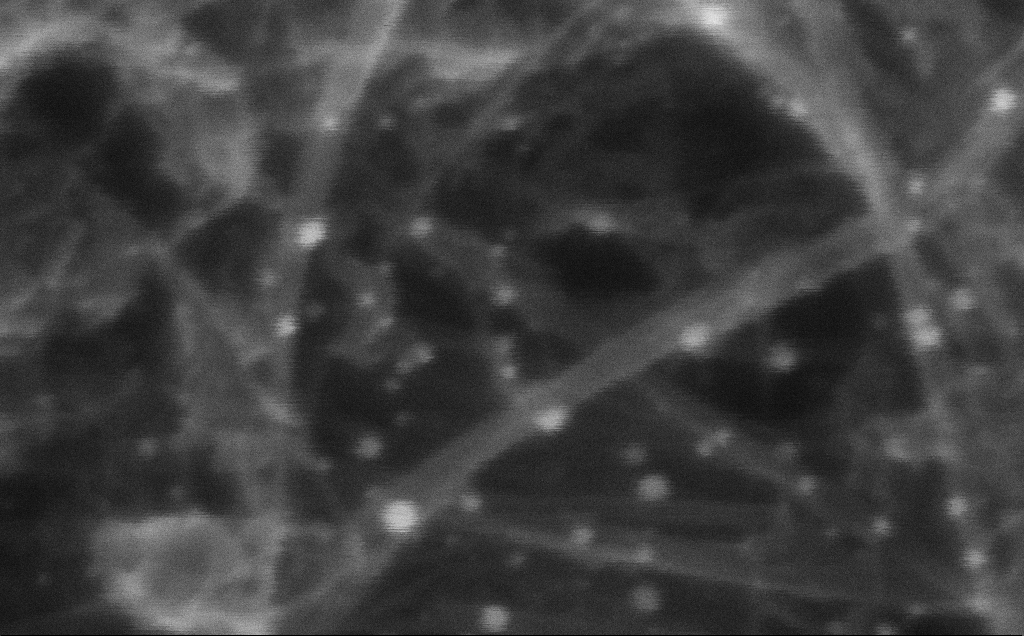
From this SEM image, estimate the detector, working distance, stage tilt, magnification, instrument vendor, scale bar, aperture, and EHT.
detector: InLens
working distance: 3 mm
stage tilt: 0°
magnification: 978.84 K X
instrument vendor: Zeiss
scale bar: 20 nm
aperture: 30 µm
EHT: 10 kV